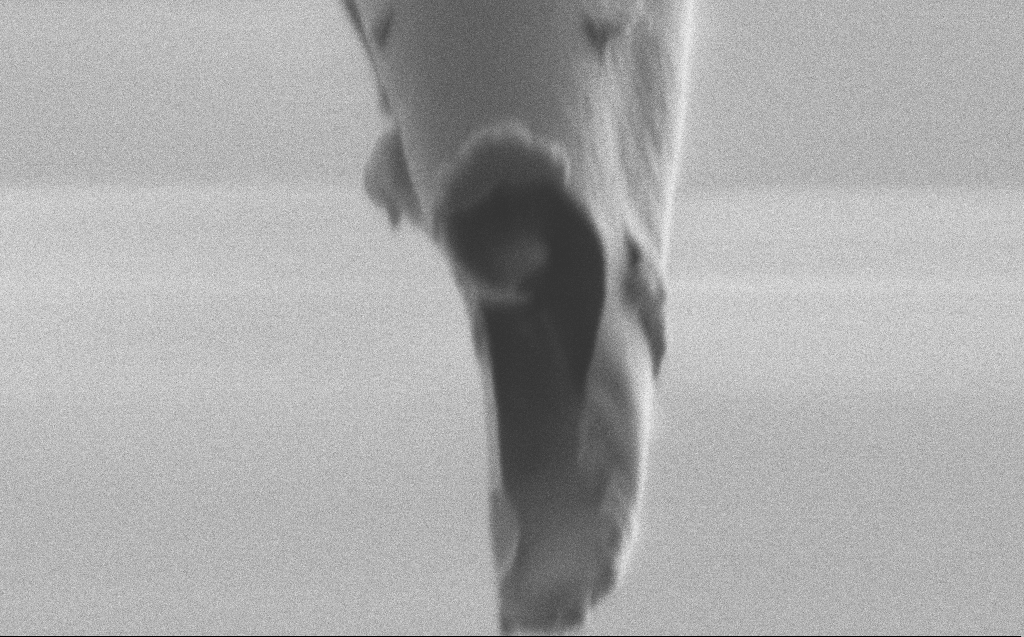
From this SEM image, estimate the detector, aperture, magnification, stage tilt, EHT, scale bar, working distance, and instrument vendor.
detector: SE2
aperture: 30 µm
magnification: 100 K X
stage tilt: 45°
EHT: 1 kV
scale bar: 200 nm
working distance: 5 mm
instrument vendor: Zeiss